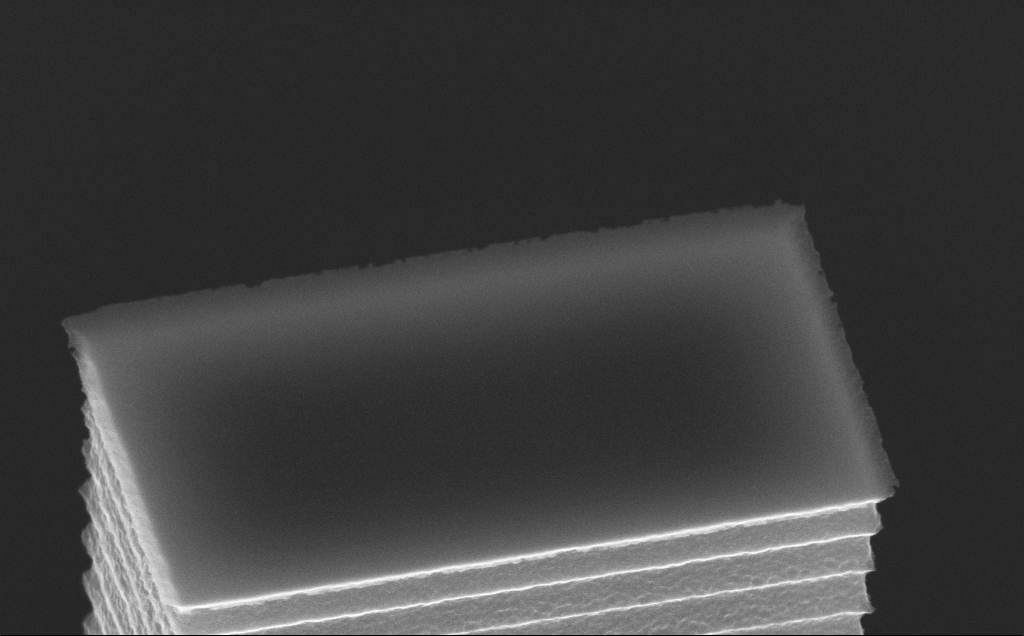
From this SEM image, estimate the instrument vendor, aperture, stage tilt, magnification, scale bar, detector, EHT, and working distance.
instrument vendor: Zeiss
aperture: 30 µm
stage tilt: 45°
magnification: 57.52 K X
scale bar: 1000 nm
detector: InLens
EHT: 10 kV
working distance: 7 mm